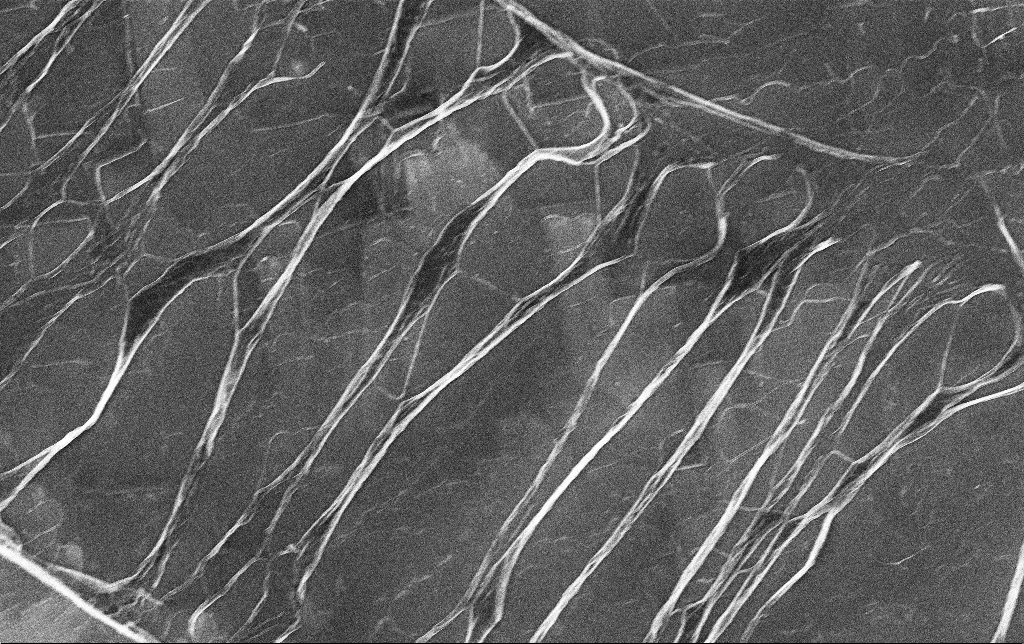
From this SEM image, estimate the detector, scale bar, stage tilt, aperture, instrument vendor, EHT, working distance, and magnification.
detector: InLens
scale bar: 10000 nm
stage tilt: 0°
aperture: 30 µm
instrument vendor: Zeiss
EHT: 5 kV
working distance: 3.8 mm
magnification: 6.99 K X